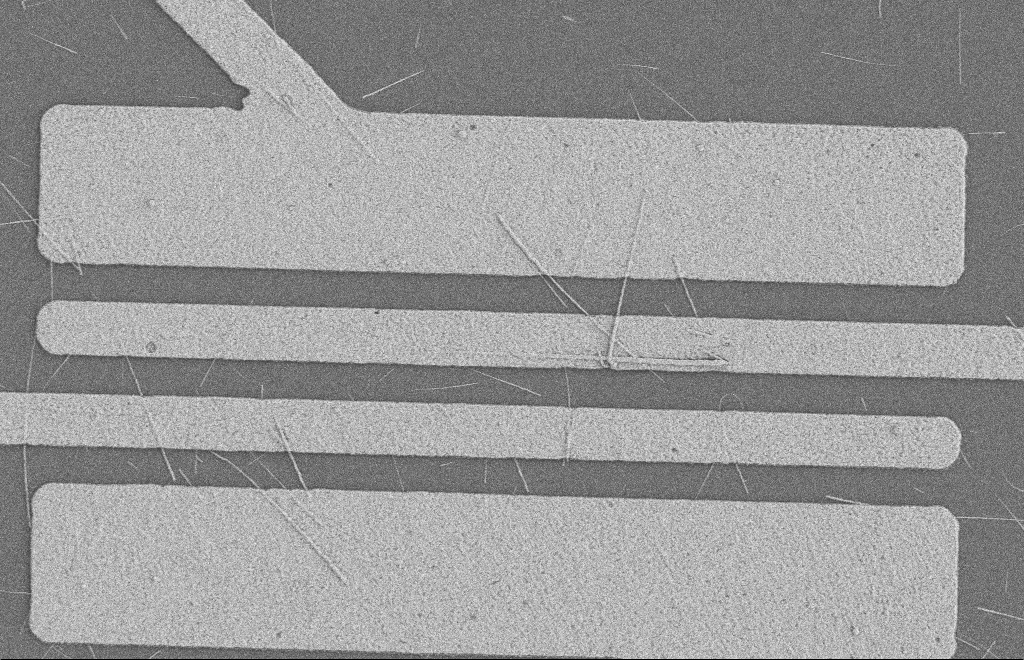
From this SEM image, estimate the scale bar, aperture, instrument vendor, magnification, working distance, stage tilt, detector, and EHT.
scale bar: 2000 nm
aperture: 20 µm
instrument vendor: Zeiss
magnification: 5.58 K X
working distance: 8 mm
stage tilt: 0°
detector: SE2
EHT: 2 kV